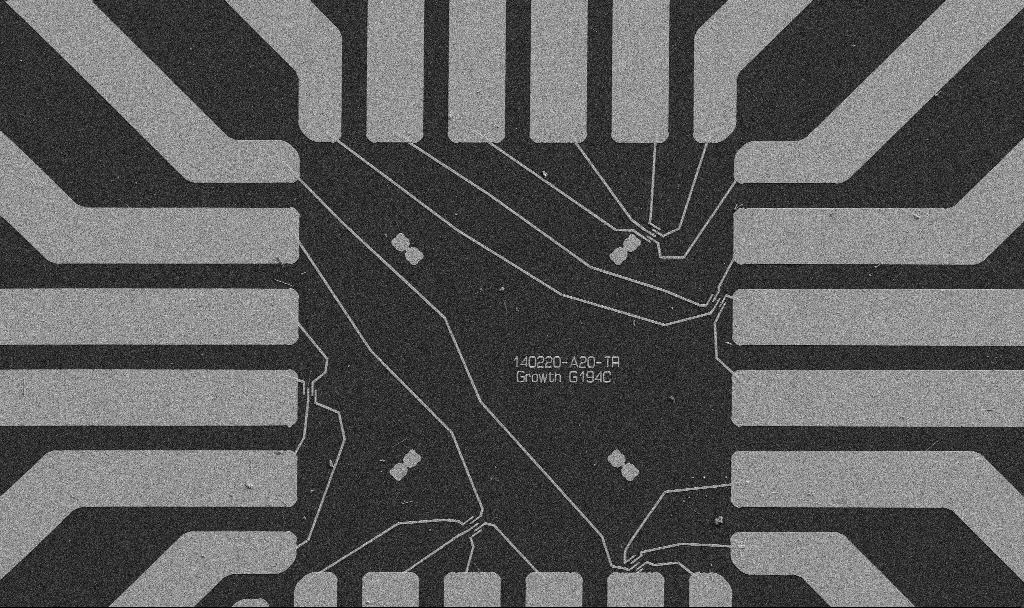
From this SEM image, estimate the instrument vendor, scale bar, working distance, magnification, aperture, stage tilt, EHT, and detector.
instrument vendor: Zeiss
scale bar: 20000 nm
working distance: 10.7 mm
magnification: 1 K X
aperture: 30 µm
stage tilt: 0°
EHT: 5 kV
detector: SE2